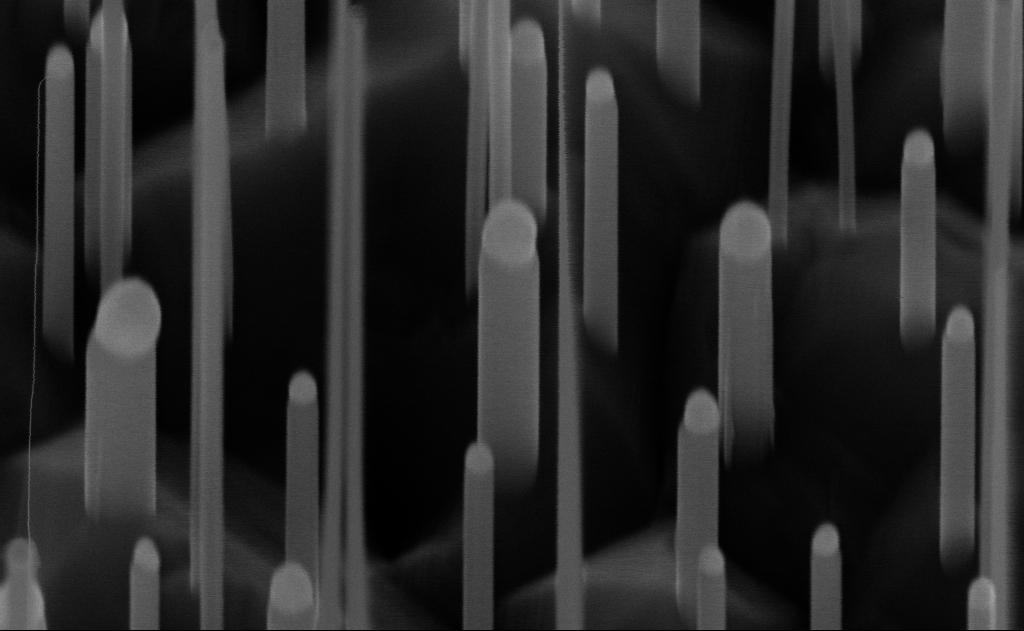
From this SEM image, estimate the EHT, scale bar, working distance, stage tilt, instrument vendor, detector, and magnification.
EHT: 10 kV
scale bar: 200 nm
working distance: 6 mm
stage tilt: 45°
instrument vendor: Zeiss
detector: InLens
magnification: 200 K X